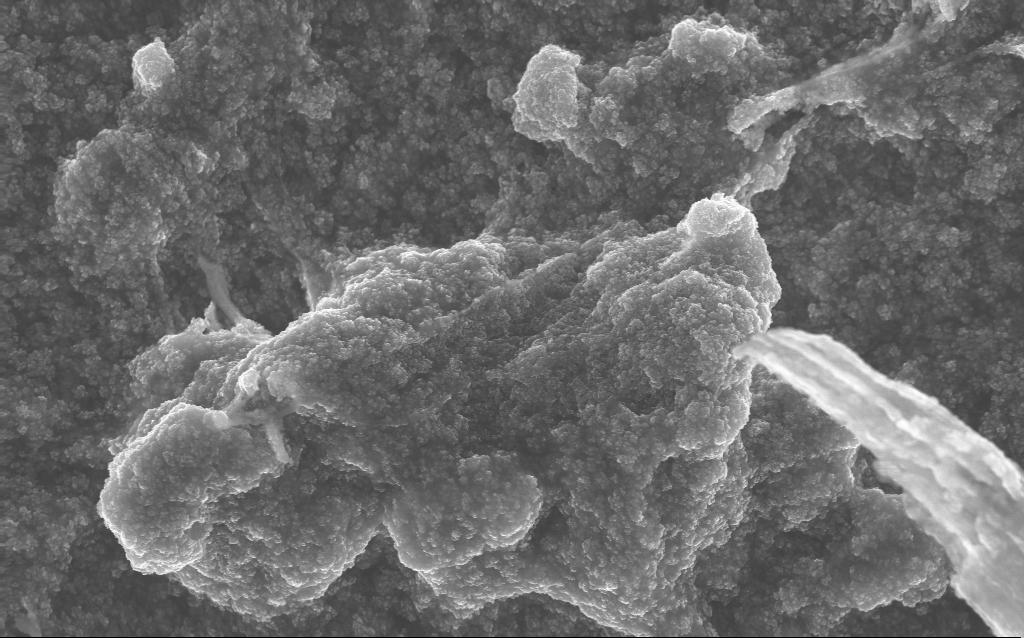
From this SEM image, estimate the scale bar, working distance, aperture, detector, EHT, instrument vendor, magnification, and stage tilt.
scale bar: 1000 nm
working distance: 2.8 mm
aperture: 30 µm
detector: InLens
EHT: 10 kV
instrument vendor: Zeiss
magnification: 20.87 K X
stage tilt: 0°